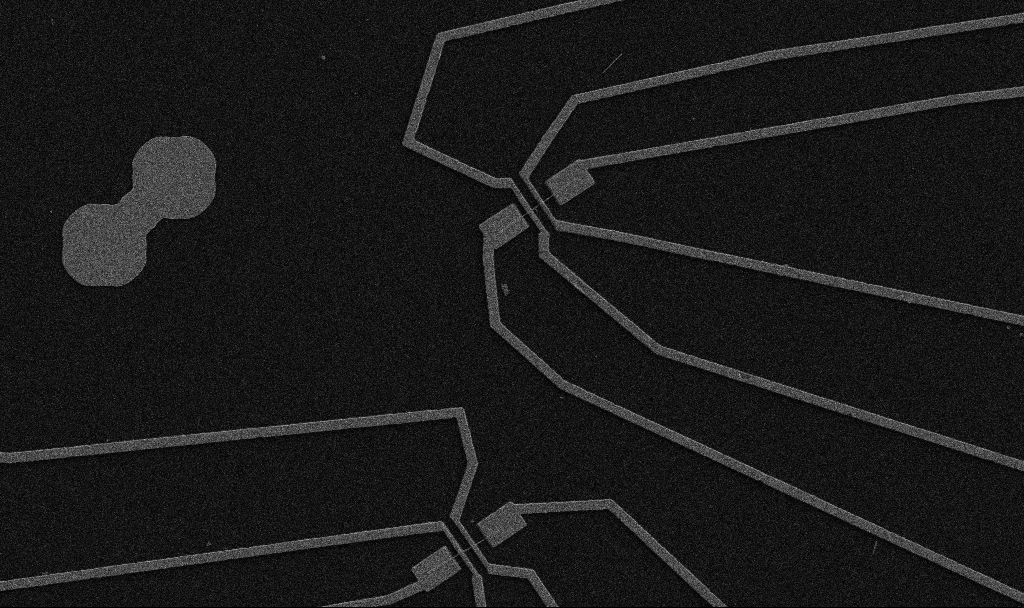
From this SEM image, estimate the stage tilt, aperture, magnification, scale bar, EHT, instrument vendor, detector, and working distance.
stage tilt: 0°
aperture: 30 µm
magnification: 5 K X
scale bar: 10000 nm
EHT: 5 kV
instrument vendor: Zeiss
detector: SE2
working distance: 10.7 mm